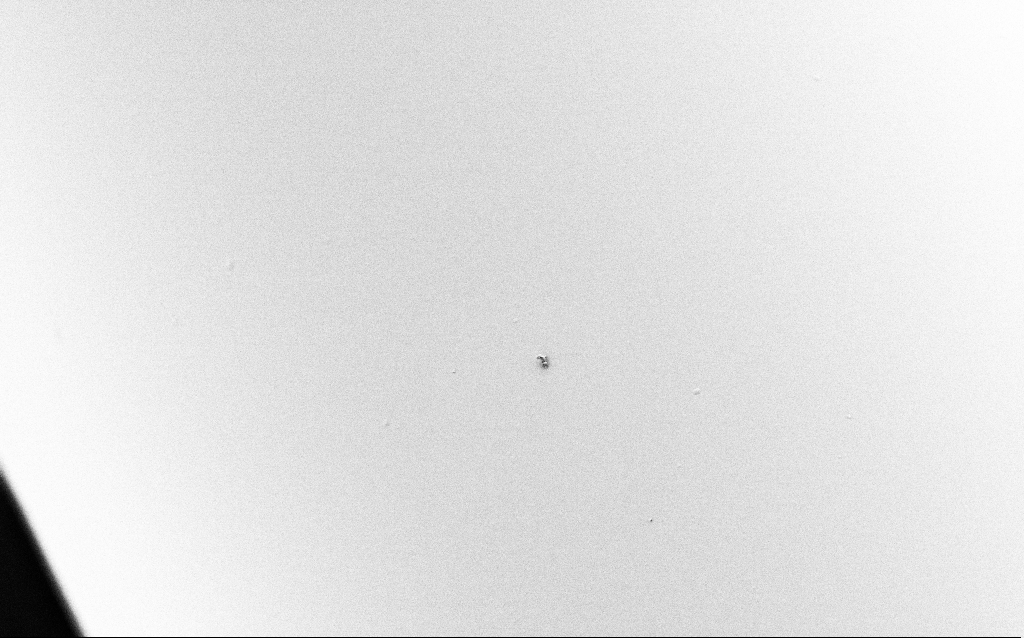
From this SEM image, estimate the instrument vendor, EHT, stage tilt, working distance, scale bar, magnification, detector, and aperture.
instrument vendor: Zeiss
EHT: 1 kV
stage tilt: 45°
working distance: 6.4 mm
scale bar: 2000 nm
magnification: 10 K X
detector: SE2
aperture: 30 µm